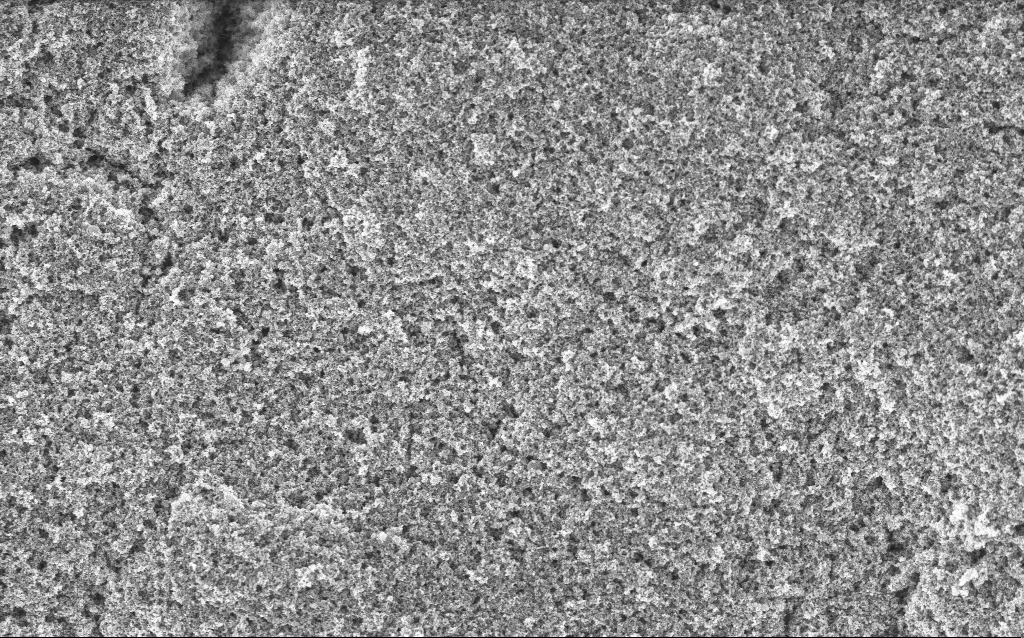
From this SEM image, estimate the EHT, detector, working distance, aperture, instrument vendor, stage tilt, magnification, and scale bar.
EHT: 5 kV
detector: InLens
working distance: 4.4 mm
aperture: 30 µm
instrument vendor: Zeiss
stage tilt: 0°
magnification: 20.87 K X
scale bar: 1000 nm